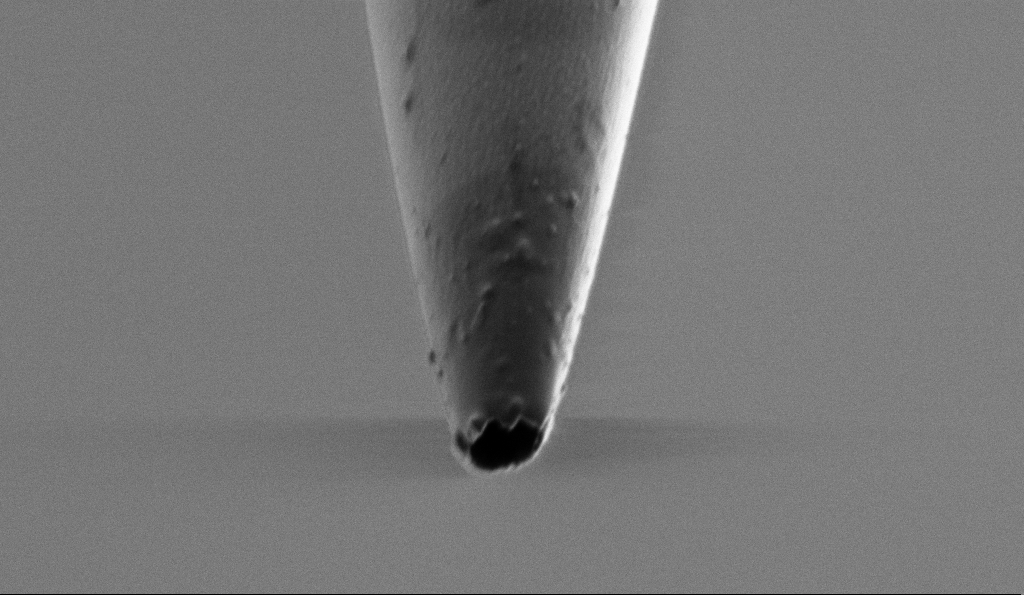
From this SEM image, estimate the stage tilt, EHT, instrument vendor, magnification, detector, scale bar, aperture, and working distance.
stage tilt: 45°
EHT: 1 kV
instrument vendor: Zeiss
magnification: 15 K X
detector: SE2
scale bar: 1000 nm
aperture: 30 µm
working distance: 6.8 mm